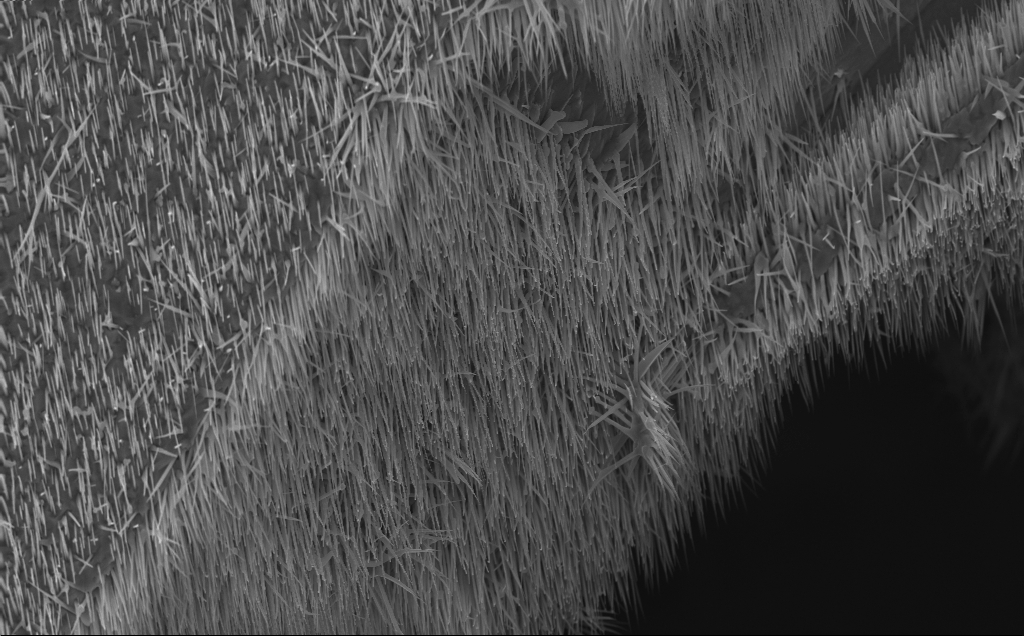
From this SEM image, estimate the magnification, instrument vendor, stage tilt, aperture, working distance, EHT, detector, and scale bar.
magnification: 7.71 K X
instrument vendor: Zeiss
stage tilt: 0°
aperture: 30 µm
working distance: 7 mm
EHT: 10 kV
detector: InLens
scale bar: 2000 nm